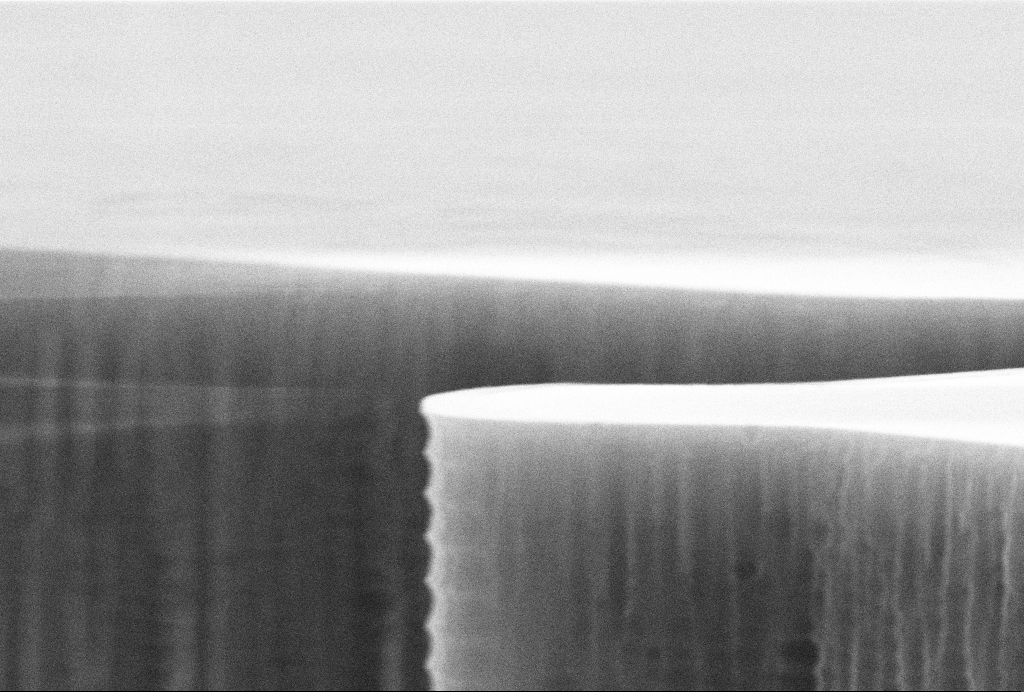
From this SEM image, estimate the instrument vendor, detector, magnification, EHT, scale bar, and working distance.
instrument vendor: Zeiss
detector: SE2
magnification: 67.03 K X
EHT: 10 kV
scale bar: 200 nm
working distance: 12.5 mm